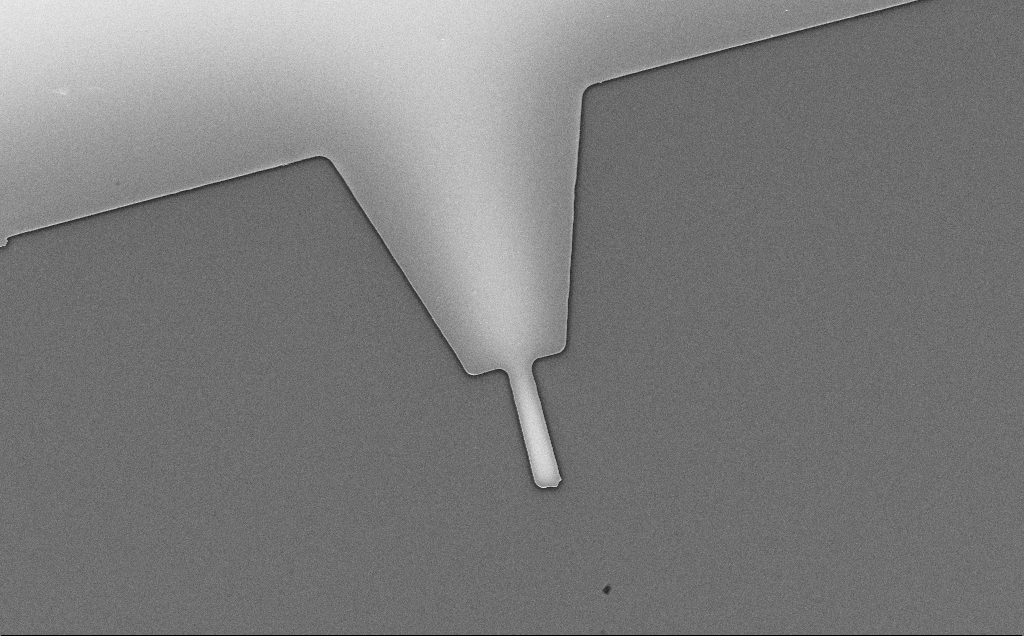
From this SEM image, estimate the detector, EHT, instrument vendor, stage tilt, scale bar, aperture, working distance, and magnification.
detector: InLens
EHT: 5 kV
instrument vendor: Zeiss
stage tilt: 0°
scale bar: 20000 nm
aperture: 30 µm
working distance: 10 mm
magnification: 1.85 K X